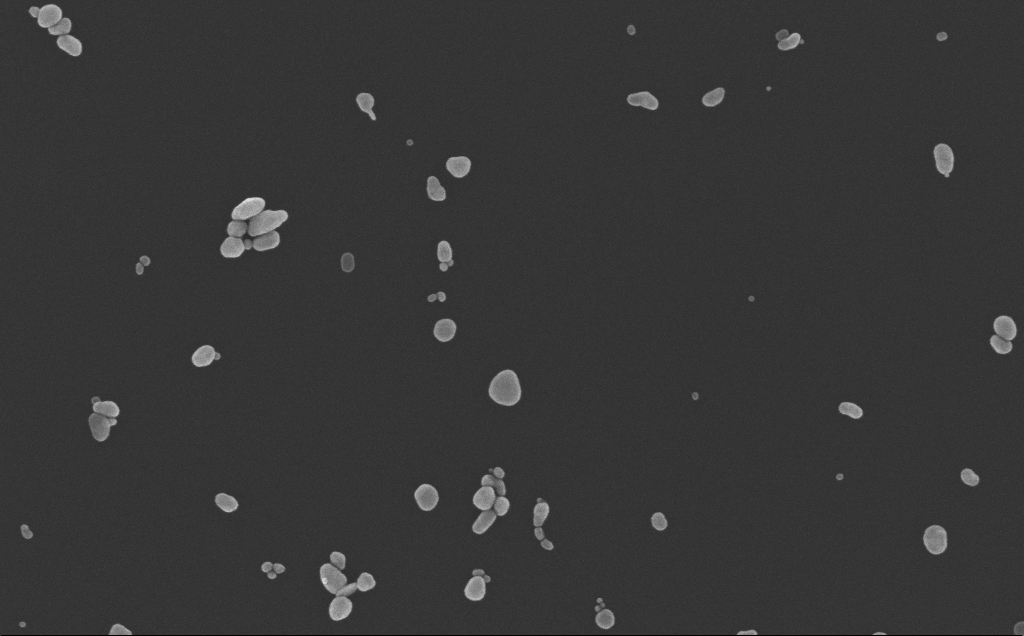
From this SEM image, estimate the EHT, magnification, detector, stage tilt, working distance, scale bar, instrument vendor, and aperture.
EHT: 10 kV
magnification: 110.93 K X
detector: InLens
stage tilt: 0°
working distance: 5 mm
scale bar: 200 nm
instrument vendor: Zeiss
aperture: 30 µm